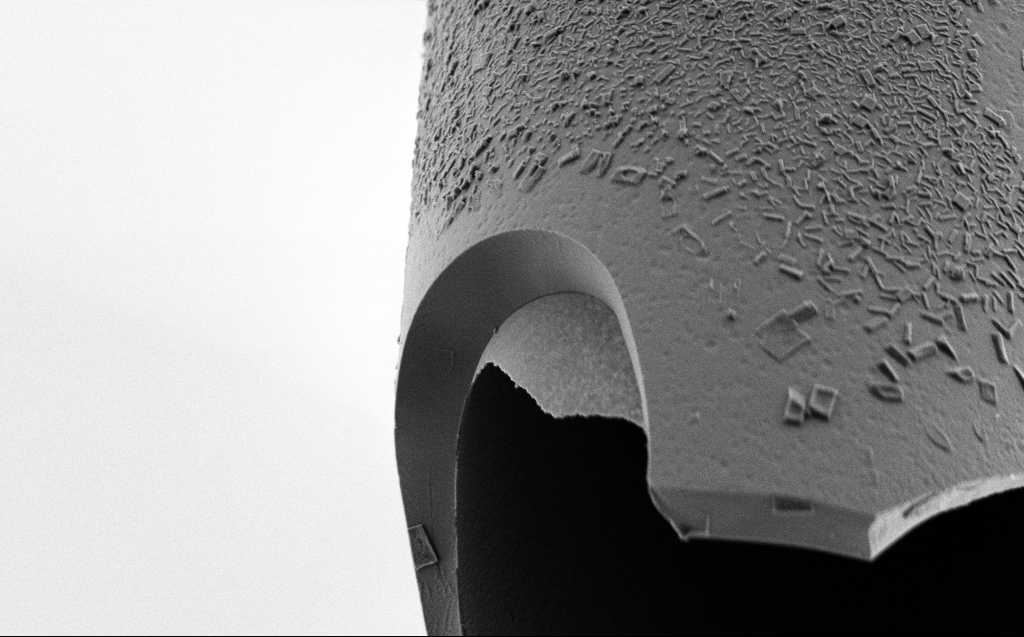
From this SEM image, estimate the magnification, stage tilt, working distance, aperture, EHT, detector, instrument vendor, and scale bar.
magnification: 11.93 K X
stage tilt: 45°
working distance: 4 mm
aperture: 30 µm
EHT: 2 kV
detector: SE2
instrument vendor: Zeiss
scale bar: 2000 nm